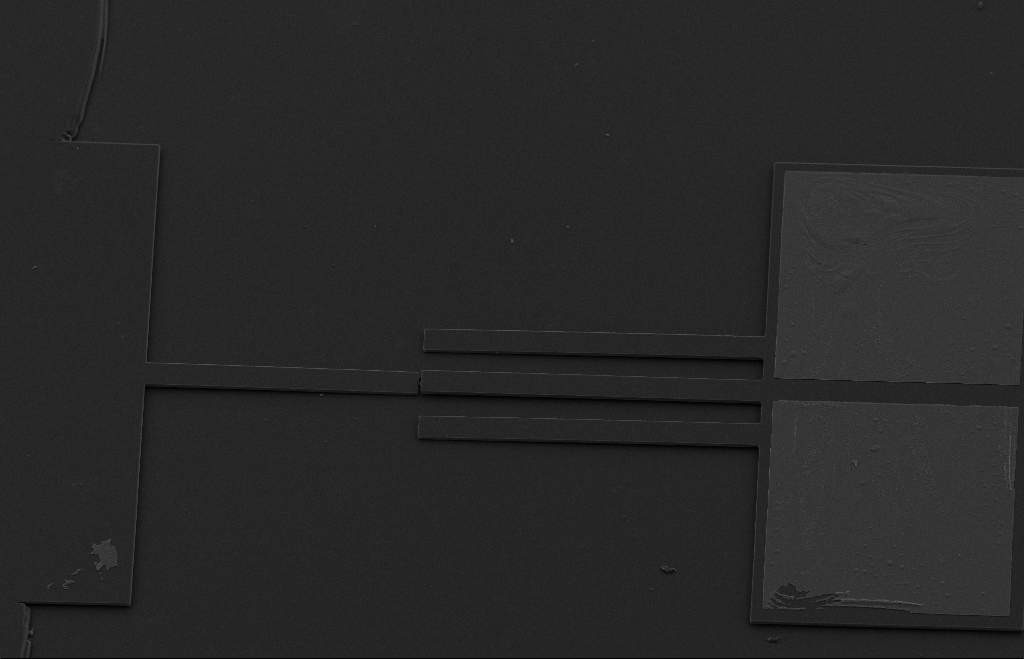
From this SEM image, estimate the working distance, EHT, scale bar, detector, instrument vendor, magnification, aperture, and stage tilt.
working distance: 9 mm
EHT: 10 kV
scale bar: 20000 nm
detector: SE2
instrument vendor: Zeiss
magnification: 0.506 K X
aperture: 20 µm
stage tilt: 33.1°